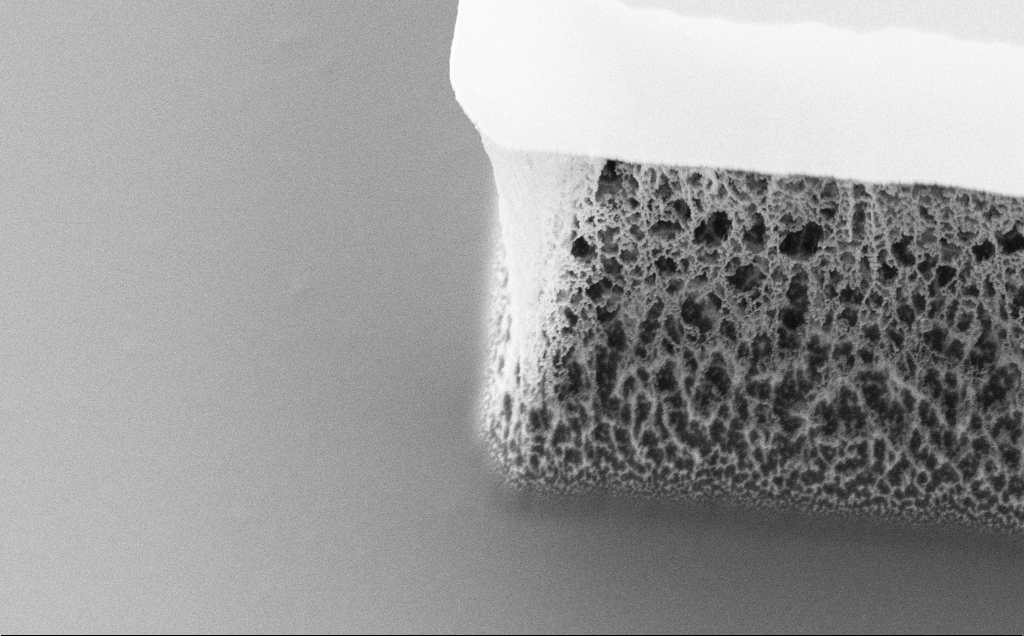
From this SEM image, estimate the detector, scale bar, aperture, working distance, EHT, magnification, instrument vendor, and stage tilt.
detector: SE2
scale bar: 2000 nm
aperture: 30 µm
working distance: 8 mm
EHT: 5 kV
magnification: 10.7 K X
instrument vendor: Zeiss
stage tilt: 45°